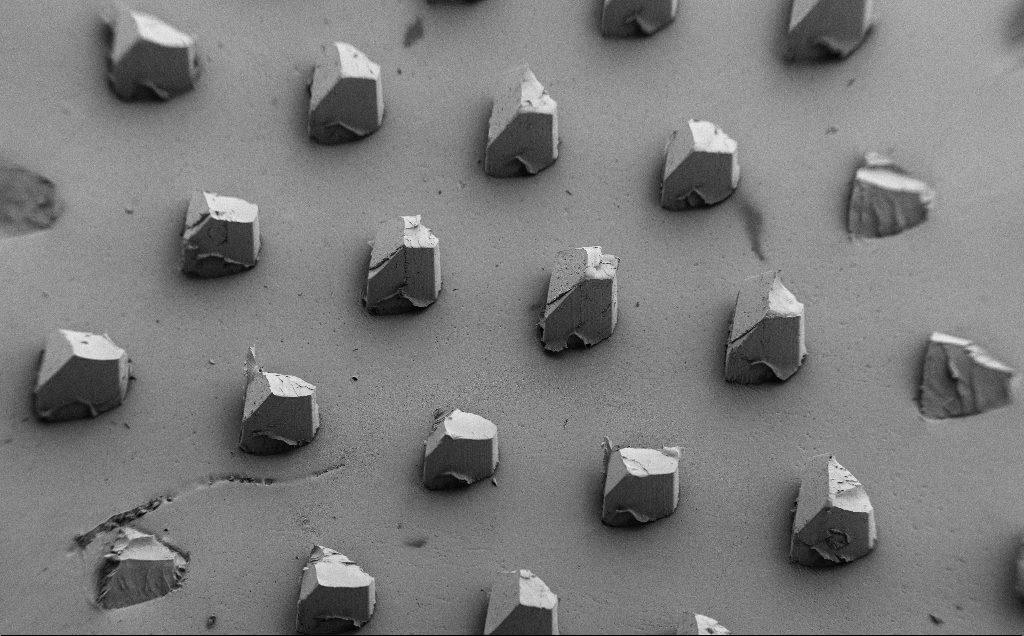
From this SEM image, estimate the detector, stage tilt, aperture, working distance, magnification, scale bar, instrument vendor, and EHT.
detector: SE2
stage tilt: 39°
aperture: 30 µm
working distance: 8 mm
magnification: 0.073 K X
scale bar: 200000 nm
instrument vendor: Zeiss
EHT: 5 kV